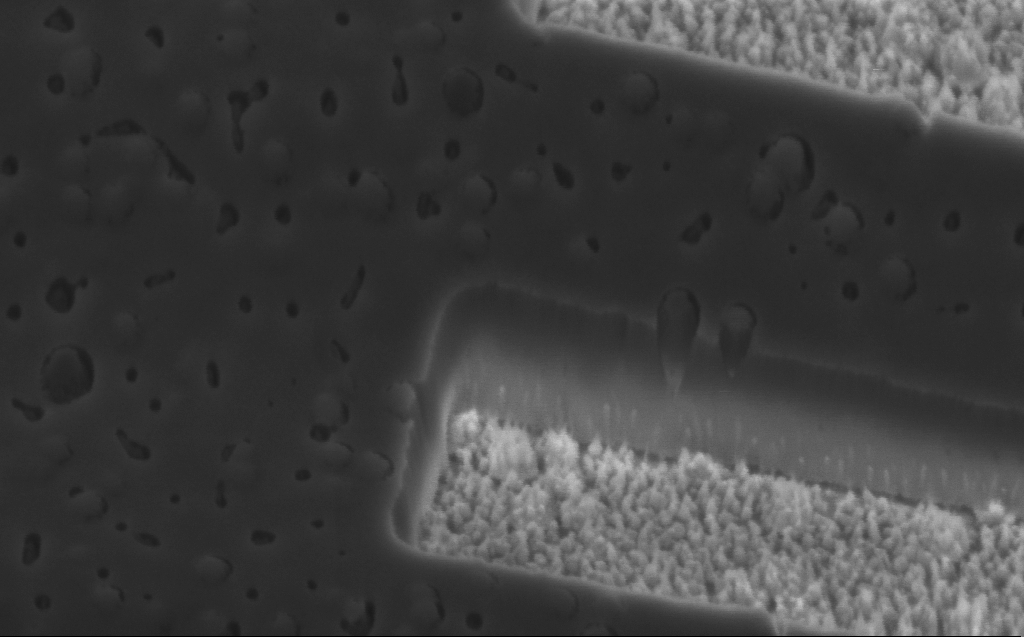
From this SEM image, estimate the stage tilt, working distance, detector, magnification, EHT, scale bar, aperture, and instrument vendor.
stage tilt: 45°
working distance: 6 mm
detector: InLens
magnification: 97.63 K X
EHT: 3 kV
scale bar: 200 nm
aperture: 30 µm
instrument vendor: Zeiss